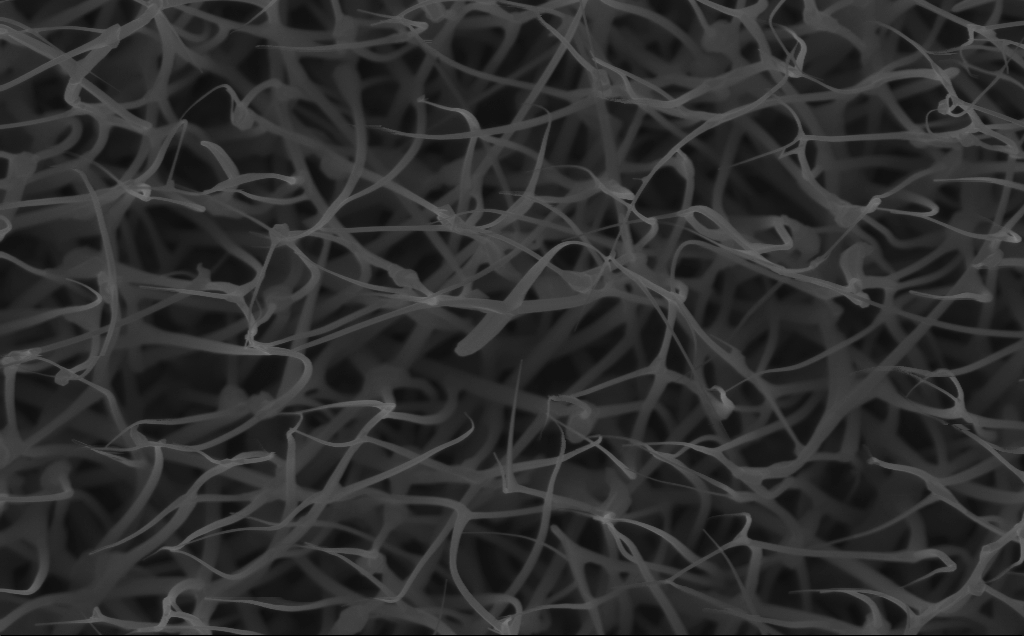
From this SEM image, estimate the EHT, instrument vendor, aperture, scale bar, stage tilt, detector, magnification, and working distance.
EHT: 10 kV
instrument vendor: Zeiss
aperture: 30 µm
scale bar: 1000 nm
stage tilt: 0°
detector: InLens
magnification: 40 K X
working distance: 6 mm